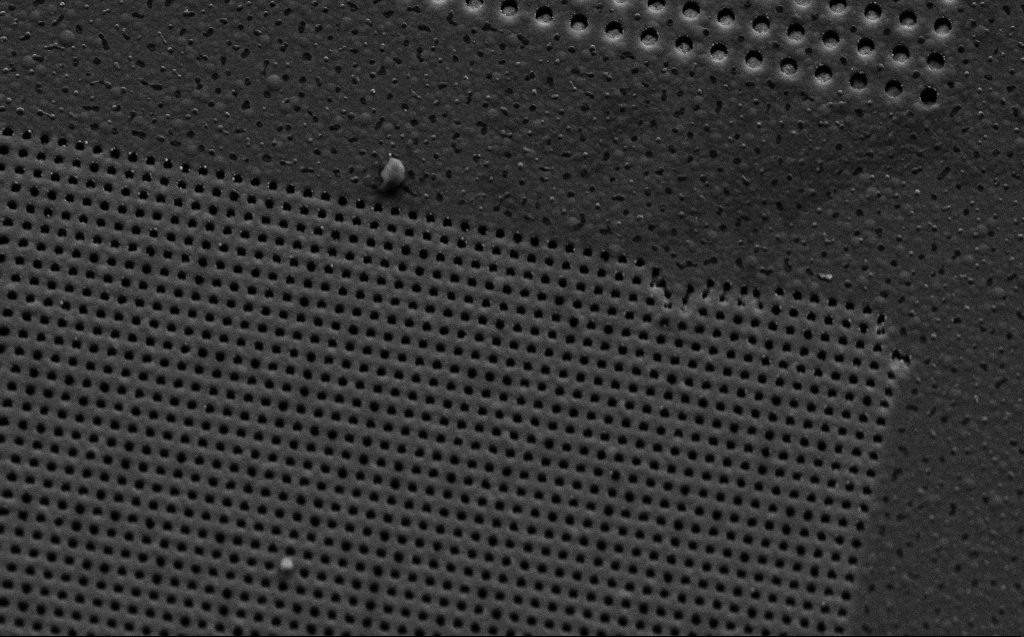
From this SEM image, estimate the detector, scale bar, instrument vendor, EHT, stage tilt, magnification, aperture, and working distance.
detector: SE2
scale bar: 1000 nm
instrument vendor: Zeiss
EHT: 5 kV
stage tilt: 45°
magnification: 16.38 K X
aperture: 30 µm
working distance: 9 mm